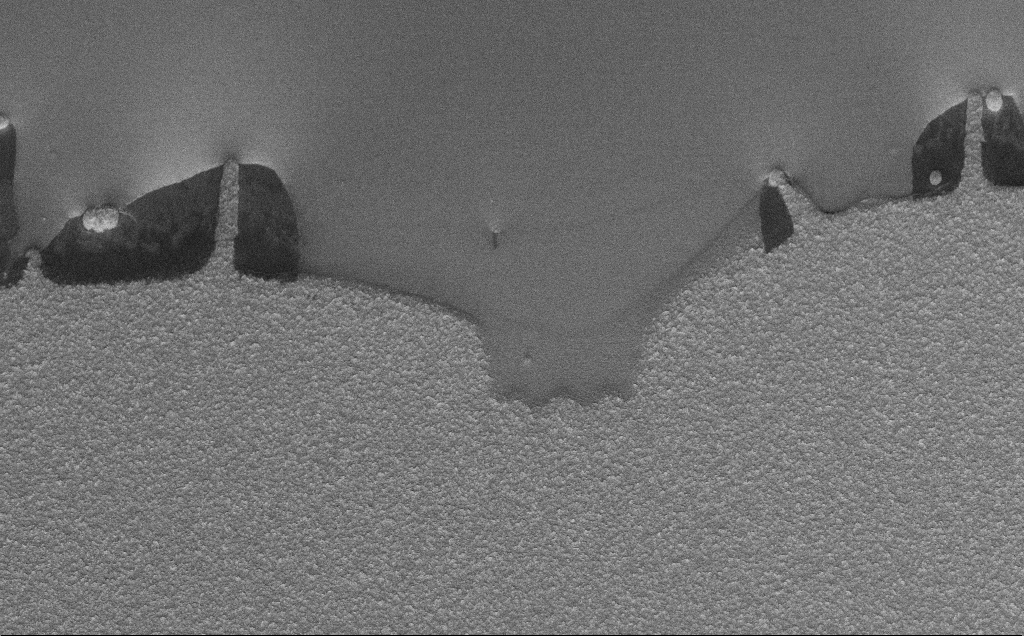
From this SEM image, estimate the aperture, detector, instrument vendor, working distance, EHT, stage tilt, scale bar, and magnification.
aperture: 30 µm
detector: InLens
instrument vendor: Zeiss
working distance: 9 mm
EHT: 10 kV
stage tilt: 45°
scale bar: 20000 nm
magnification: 1.62 K X